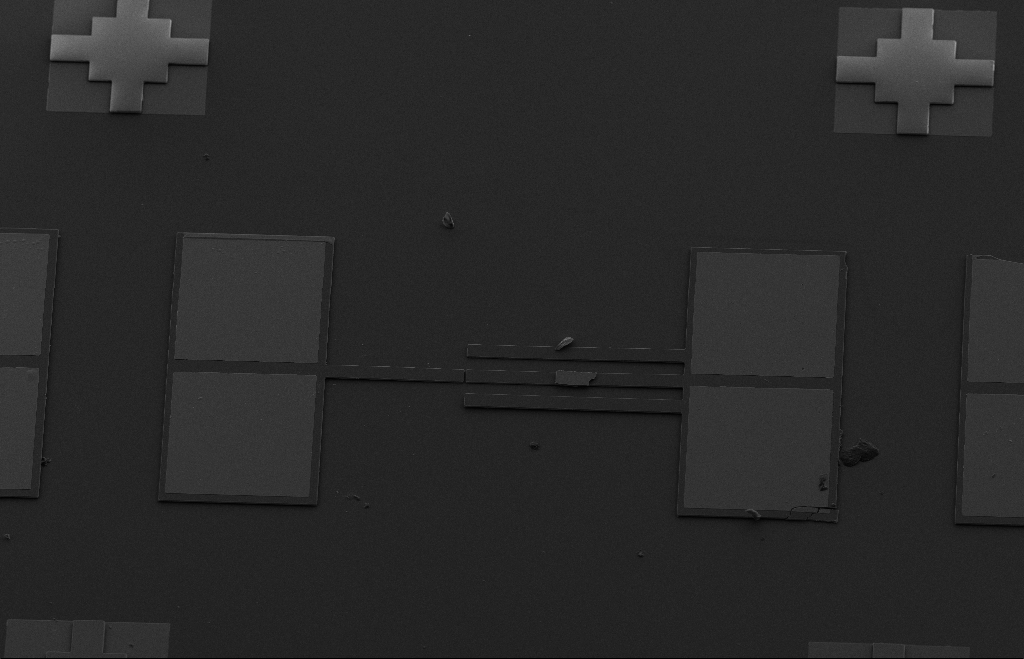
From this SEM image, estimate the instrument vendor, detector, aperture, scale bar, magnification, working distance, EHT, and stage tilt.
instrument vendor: Zeiss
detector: SE2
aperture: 20 µm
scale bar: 100000 nm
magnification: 0.294 K X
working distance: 9 mm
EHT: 10 kV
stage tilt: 33.1°